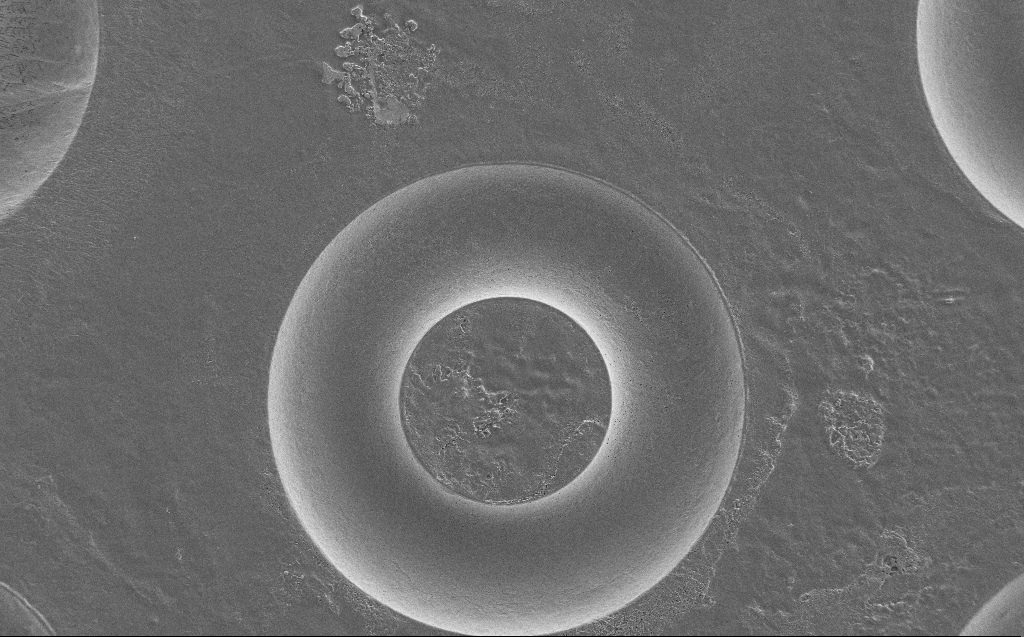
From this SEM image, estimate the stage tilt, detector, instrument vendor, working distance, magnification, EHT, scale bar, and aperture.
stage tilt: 0°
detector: SE2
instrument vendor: Zeiss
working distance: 7 mm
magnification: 0.484 K X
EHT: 2 kV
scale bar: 100000 nm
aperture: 30 µm